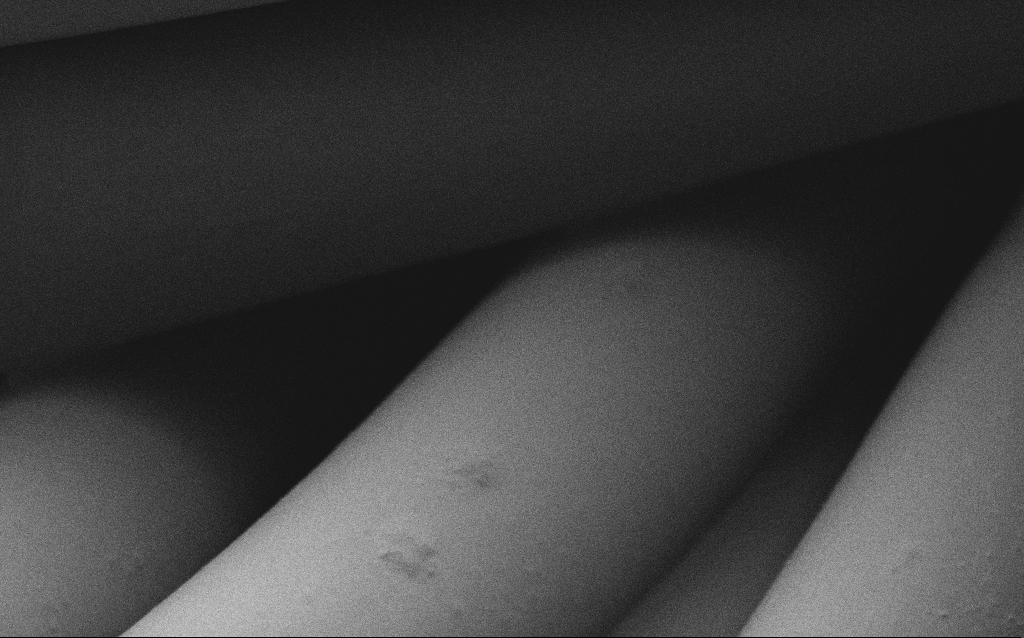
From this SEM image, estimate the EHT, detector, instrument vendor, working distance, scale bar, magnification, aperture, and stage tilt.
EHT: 1 kV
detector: SE2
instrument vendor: Zeiss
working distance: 4 mm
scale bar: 10000 nm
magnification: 5.15 K X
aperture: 30 µm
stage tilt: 0°